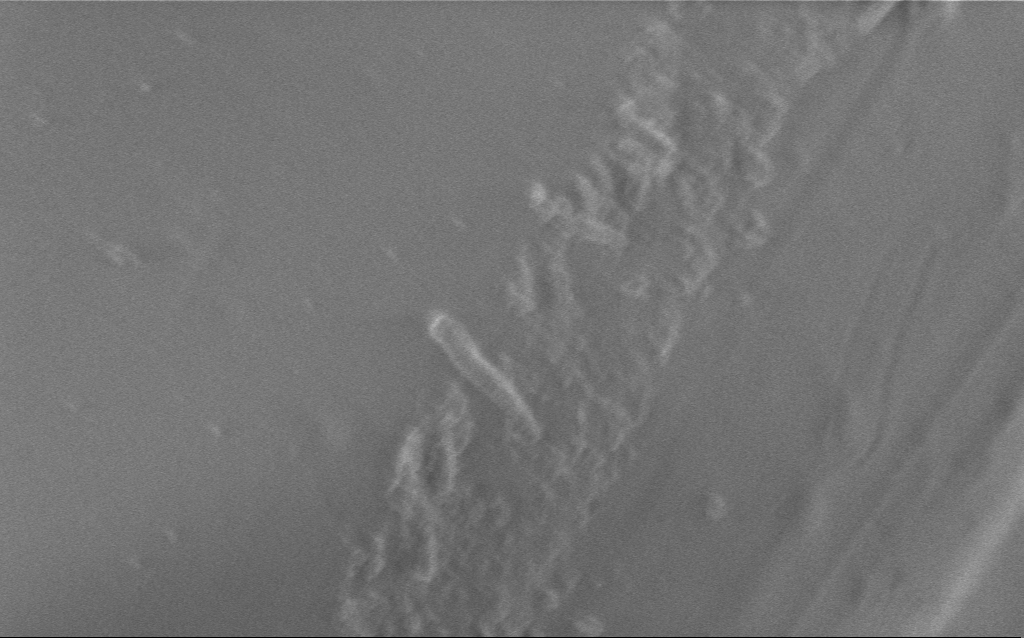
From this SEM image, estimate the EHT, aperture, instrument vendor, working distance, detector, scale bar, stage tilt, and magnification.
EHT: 1 kV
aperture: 30 µm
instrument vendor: Zeiss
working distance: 4 mm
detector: InLens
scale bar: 2000 nm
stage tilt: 0°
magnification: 23.69 K X